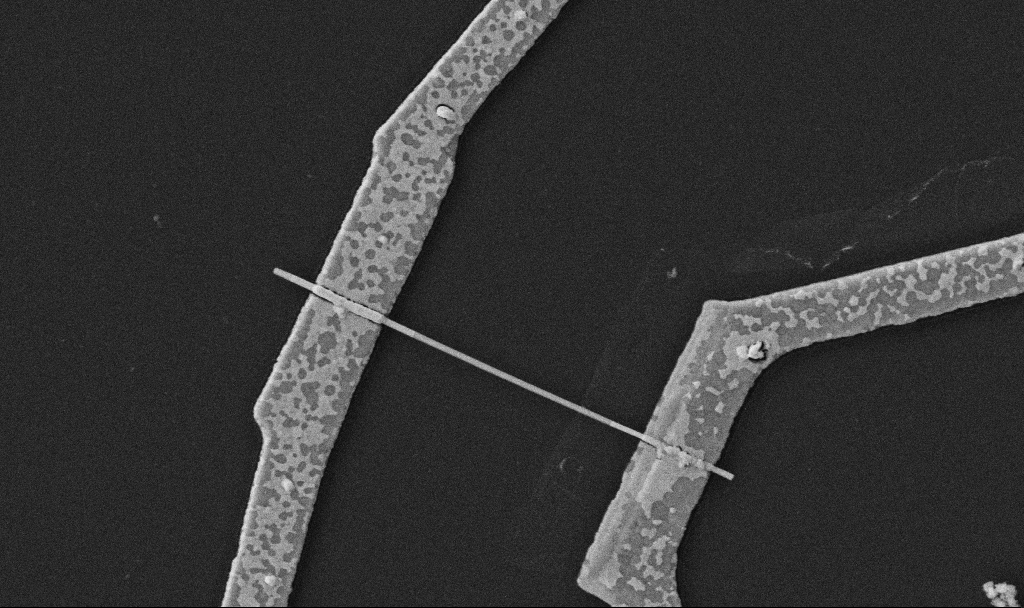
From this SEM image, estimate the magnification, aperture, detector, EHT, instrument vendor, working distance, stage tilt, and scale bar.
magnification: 30 K X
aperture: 30 µm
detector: SE2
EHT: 5 kV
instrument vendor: Zeiss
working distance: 10.7 mm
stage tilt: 0°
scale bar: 1000 nm